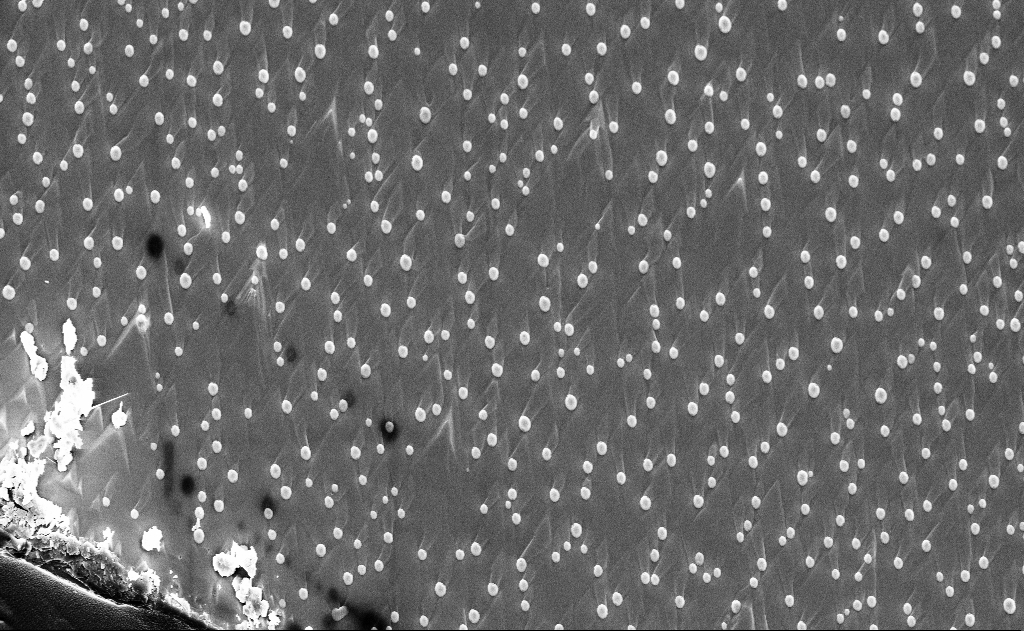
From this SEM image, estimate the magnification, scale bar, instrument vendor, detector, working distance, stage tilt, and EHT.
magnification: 5 K X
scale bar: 10000 nm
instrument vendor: Zeiss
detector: InLens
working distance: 12 mm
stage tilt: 0°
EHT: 10 kV